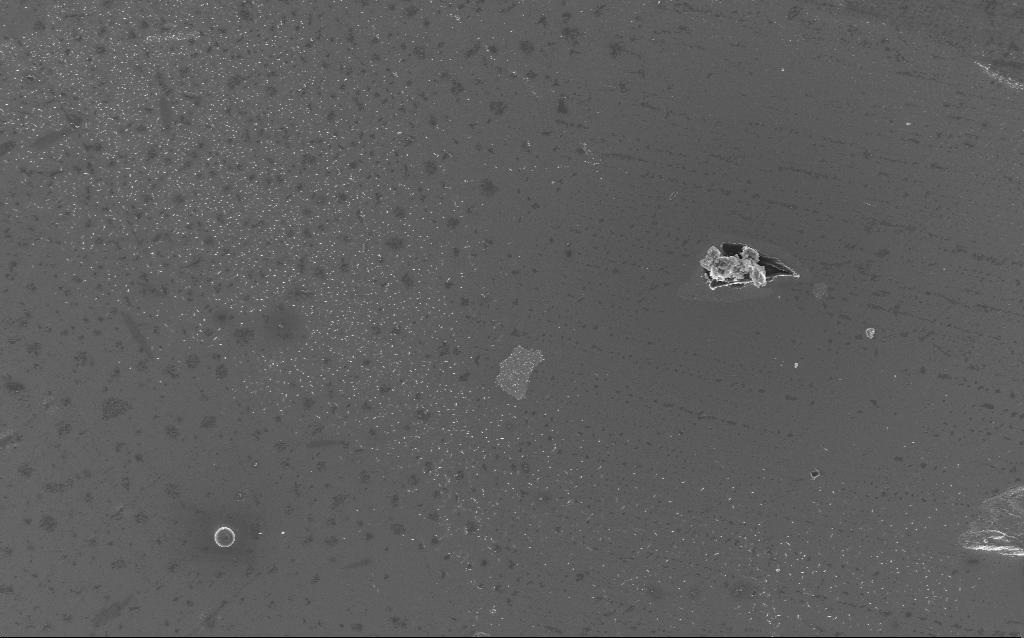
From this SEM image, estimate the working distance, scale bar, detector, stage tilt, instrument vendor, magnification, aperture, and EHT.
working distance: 2 mm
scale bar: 10000 nm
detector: InLens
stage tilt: -0°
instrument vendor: Zeiss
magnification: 1.97 K X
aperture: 30 µm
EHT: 5 kV